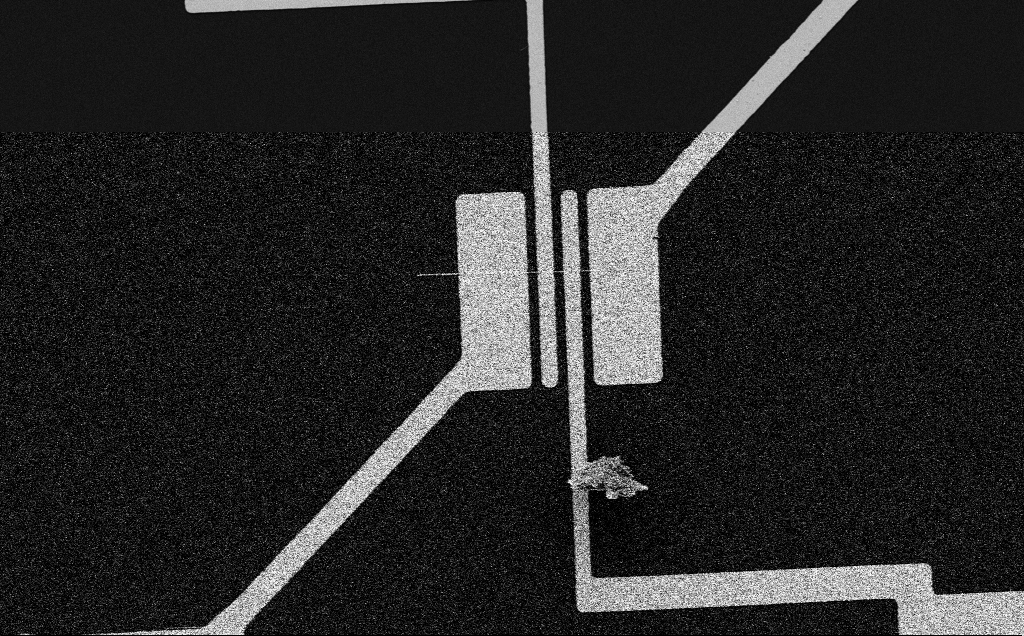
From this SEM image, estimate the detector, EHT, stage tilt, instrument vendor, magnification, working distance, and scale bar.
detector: SE2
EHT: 5 kV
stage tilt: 0°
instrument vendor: Zeiss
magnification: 2.41 K X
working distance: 10 mm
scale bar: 20000 nm